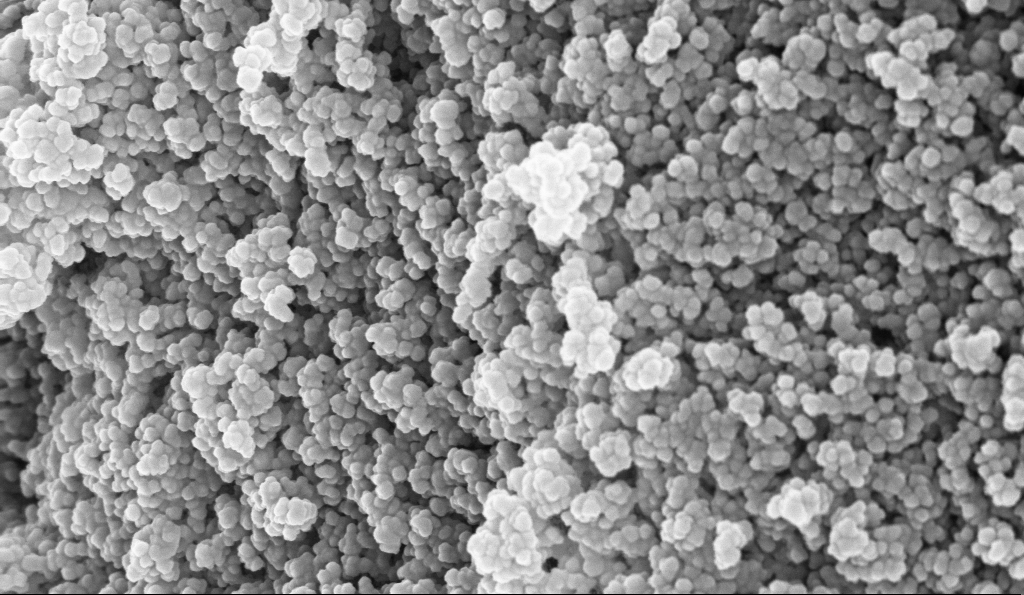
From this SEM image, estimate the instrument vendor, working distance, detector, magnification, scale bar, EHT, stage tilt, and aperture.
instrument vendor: Zeiss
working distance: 5.1 mm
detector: InLens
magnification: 135 K X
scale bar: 100 nm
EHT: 10 kV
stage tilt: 0°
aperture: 30 µm